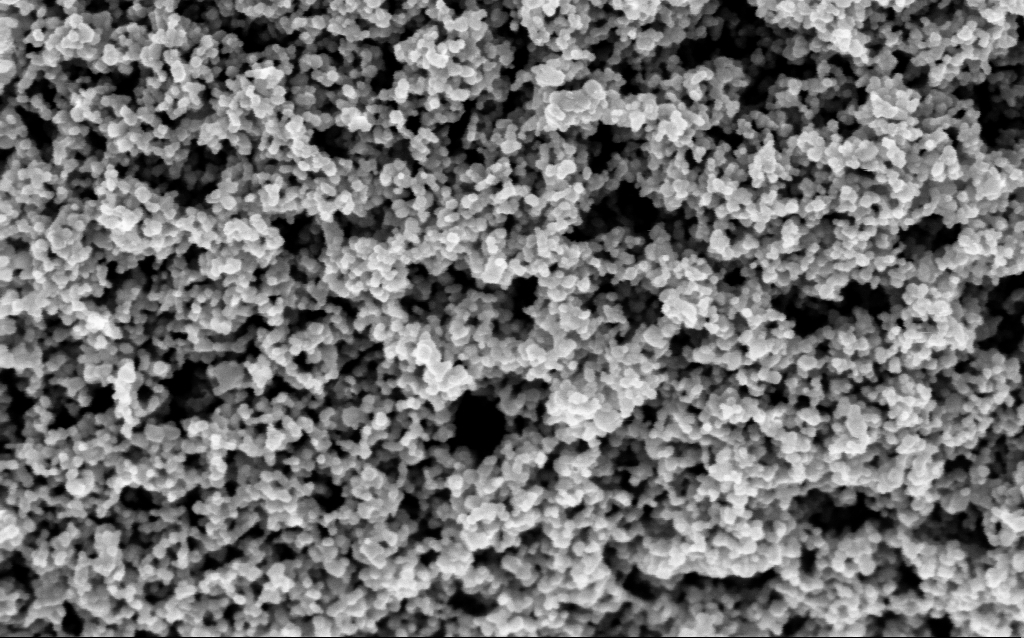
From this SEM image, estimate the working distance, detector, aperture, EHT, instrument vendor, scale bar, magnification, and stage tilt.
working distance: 7.5 mm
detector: InLens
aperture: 30 µm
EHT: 3 kV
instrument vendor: Zeiss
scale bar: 100 nm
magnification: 130 K X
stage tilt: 0°